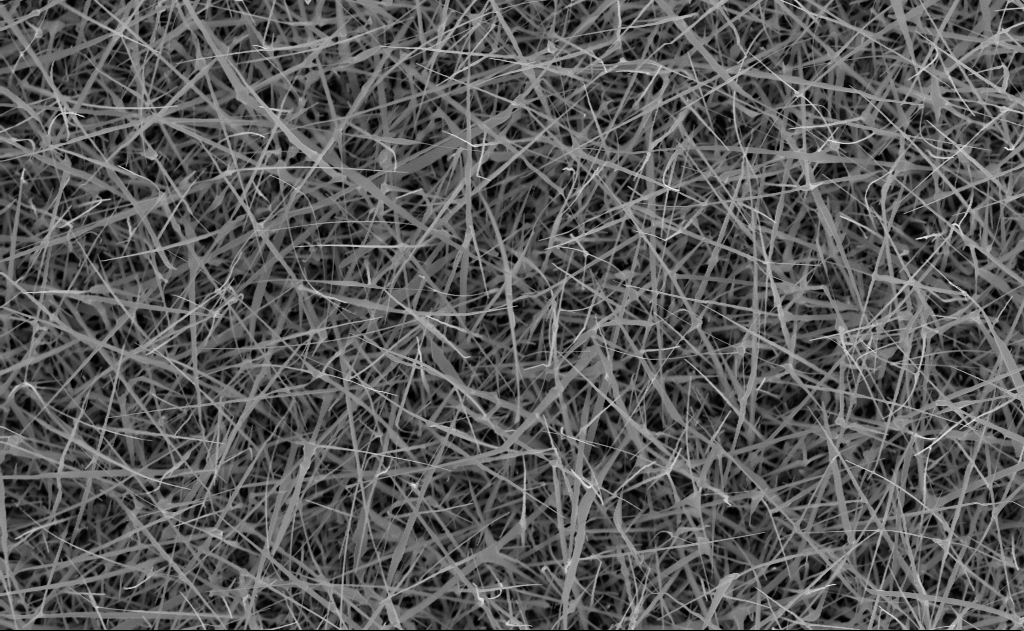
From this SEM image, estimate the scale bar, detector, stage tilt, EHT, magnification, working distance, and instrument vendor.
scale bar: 1000 nm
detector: InLens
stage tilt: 0°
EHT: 10 kV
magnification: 20 K X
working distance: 10 mm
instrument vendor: Zeiss